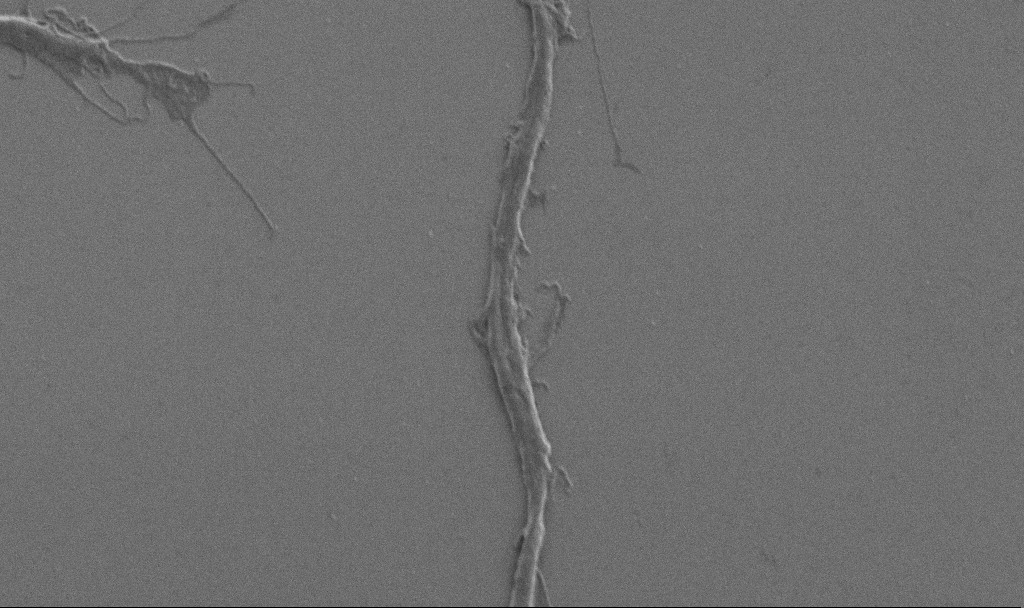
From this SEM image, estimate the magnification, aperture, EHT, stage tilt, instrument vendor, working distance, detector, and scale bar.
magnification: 10 K X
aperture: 30 µm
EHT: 1 kV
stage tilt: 0°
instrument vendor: Zeiss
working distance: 6.9 mm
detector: SE2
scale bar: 2000 nm